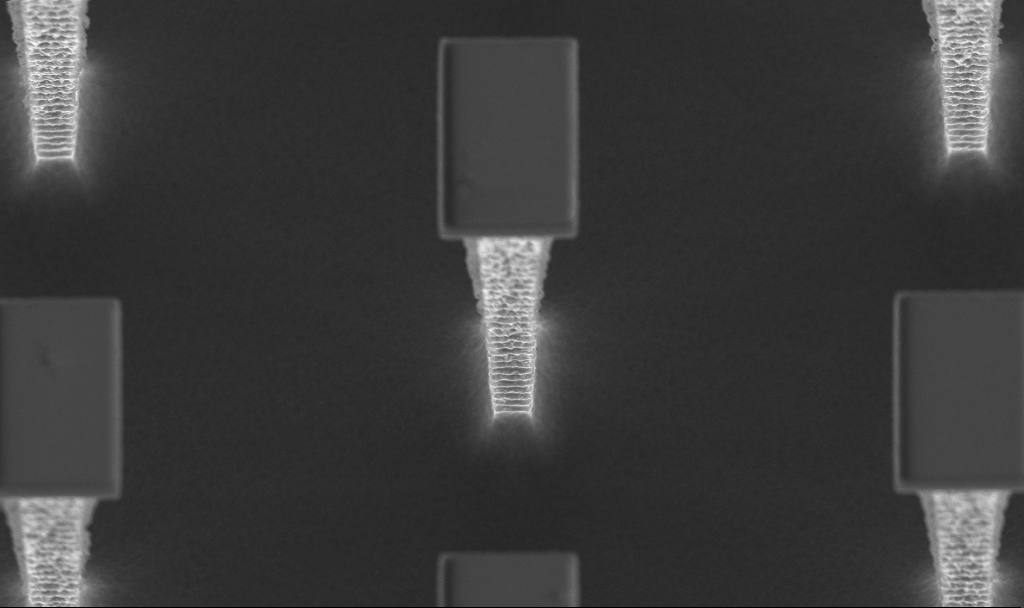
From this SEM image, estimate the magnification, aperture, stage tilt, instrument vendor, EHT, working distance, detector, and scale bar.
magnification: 16.87 K X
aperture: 30 µm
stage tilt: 20°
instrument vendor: Zeiss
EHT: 5 kV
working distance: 4.2 mm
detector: InLens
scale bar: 1000 nm